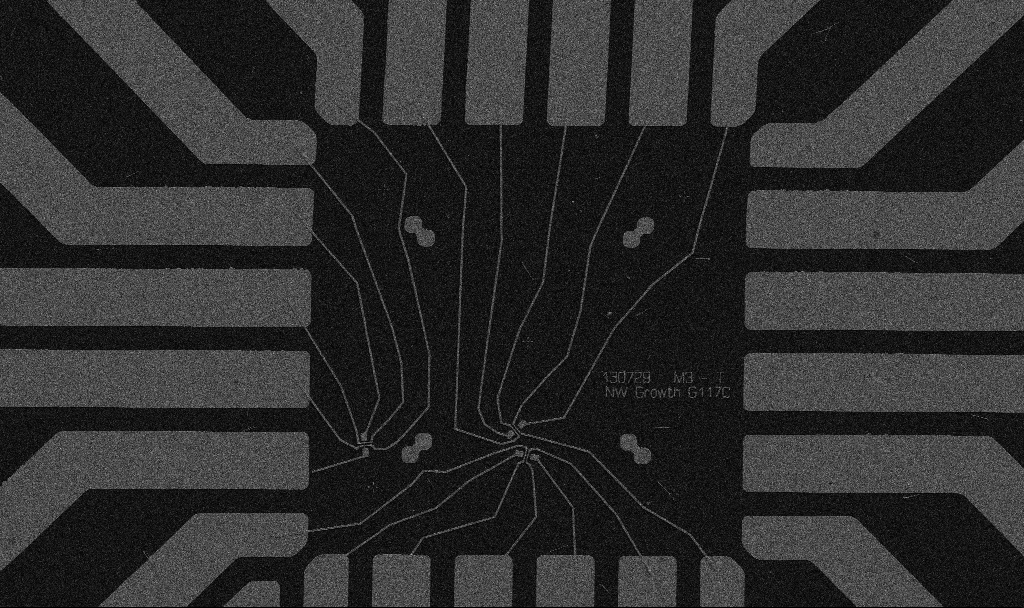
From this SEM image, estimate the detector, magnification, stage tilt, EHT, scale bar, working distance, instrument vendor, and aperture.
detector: SE2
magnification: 1 K X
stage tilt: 0°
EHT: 5 kV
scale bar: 20000 nm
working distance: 10.7 mm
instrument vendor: Zeiss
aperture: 30 µm